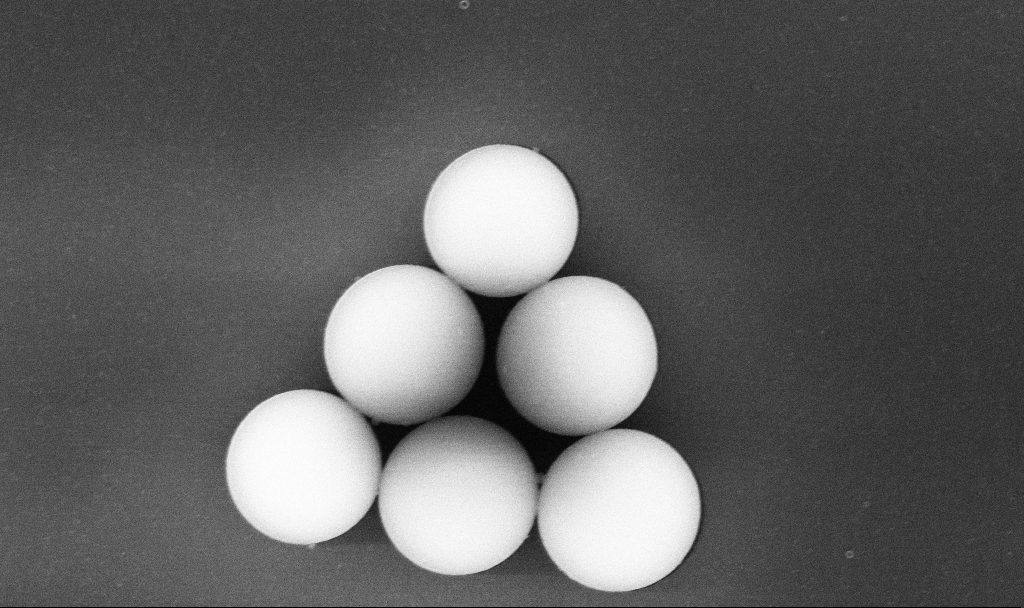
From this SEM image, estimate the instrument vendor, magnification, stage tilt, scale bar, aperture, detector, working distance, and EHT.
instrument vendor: Zeiss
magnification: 28.36 K X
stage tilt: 0°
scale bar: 2000 nm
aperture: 30 µm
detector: InLens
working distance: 5.2 mm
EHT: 10 kV